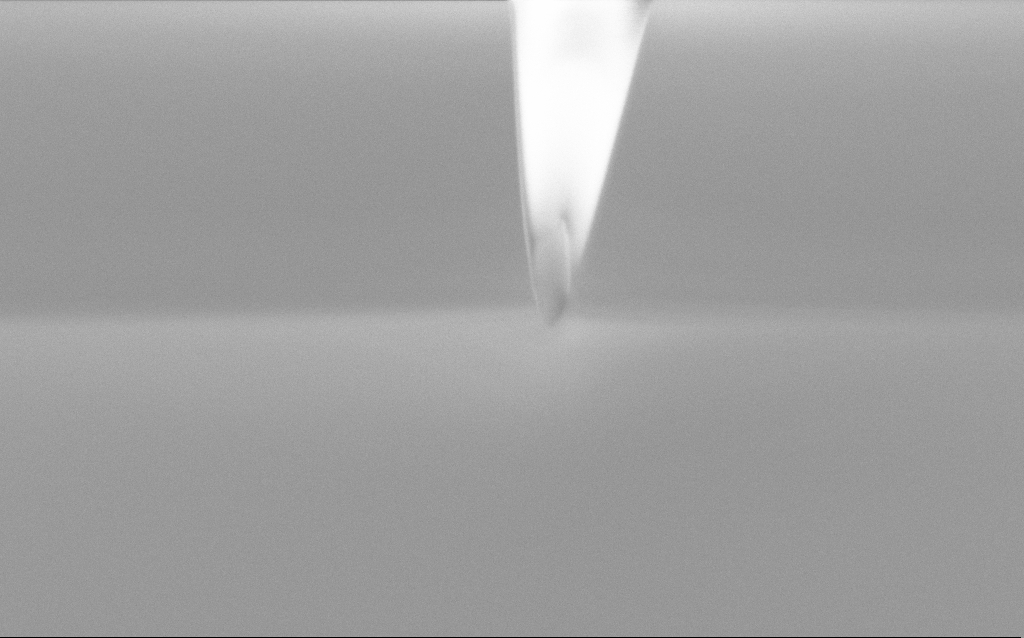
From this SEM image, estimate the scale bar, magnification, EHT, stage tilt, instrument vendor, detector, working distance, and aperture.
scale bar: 1000 nm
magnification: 57.83 K X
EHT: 2 kV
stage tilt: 45°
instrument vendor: Zeiss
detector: InLens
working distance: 5 mm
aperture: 30 µm